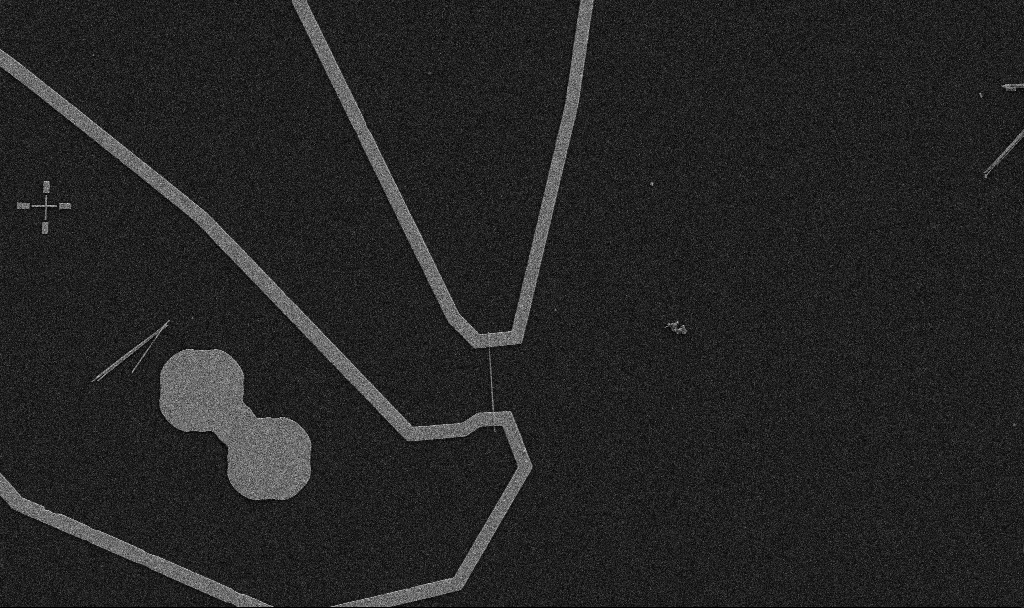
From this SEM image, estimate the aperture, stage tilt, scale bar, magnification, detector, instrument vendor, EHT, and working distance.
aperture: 30 µm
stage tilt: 0°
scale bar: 10000 nm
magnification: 5 K X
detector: SE2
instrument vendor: Zeiss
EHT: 5 kV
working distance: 10.7 mm